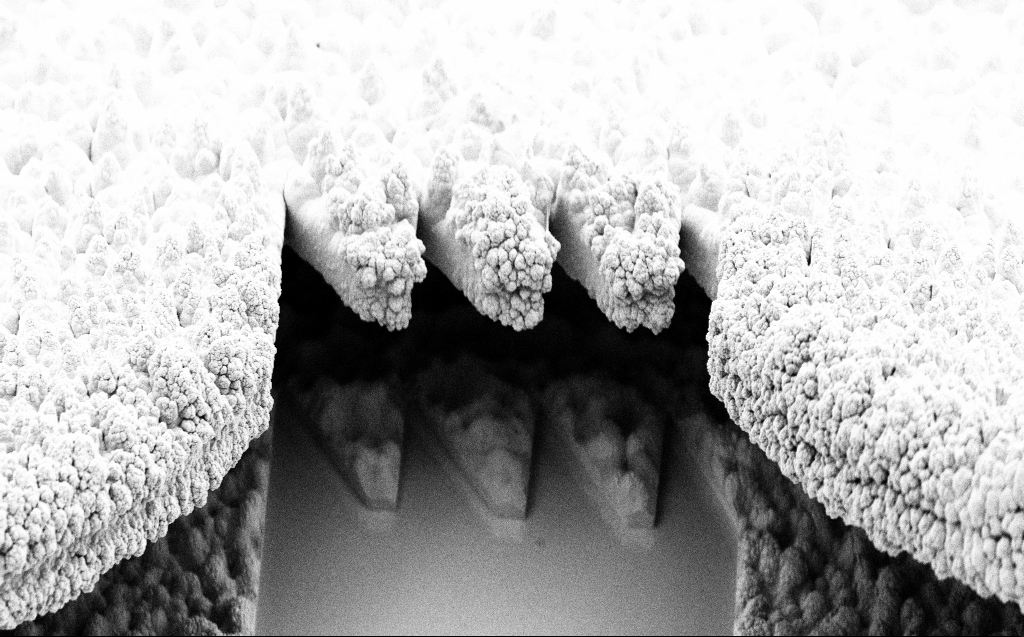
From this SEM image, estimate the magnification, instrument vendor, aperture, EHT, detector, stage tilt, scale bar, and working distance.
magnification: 4.2 K X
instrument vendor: Zeiss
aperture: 30 µm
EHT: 5 kV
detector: SE2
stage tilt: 45.1°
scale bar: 10000 nm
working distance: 9 mm